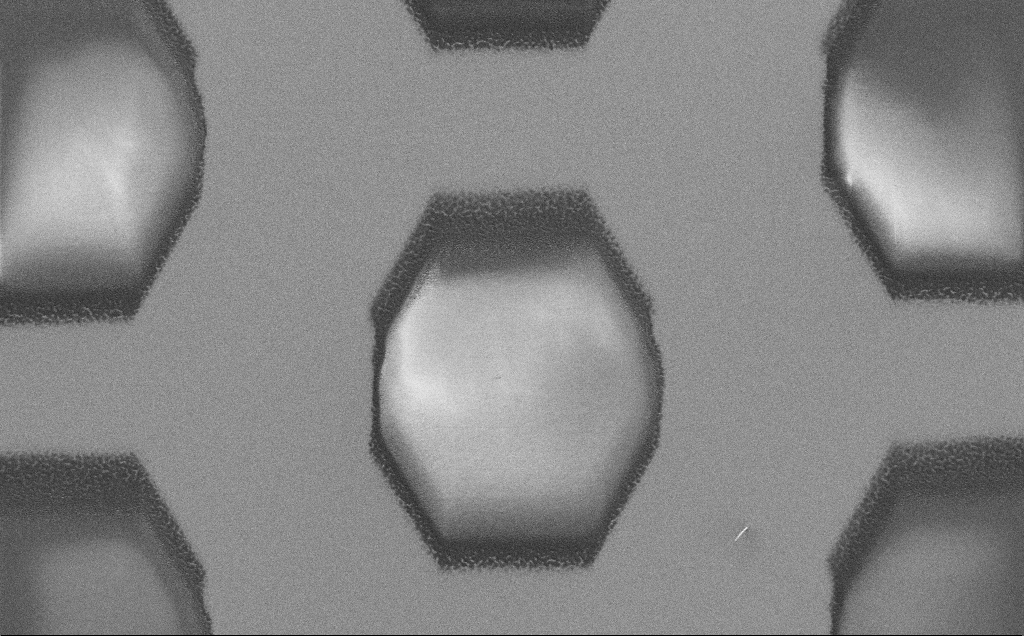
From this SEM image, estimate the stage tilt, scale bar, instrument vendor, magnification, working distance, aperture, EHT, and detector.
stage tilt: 0°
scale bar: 10000 nm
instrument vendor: Zeiss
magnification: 1.92 K X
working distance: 6 mm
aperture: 30 µm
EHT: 1.5 kV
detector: SE2